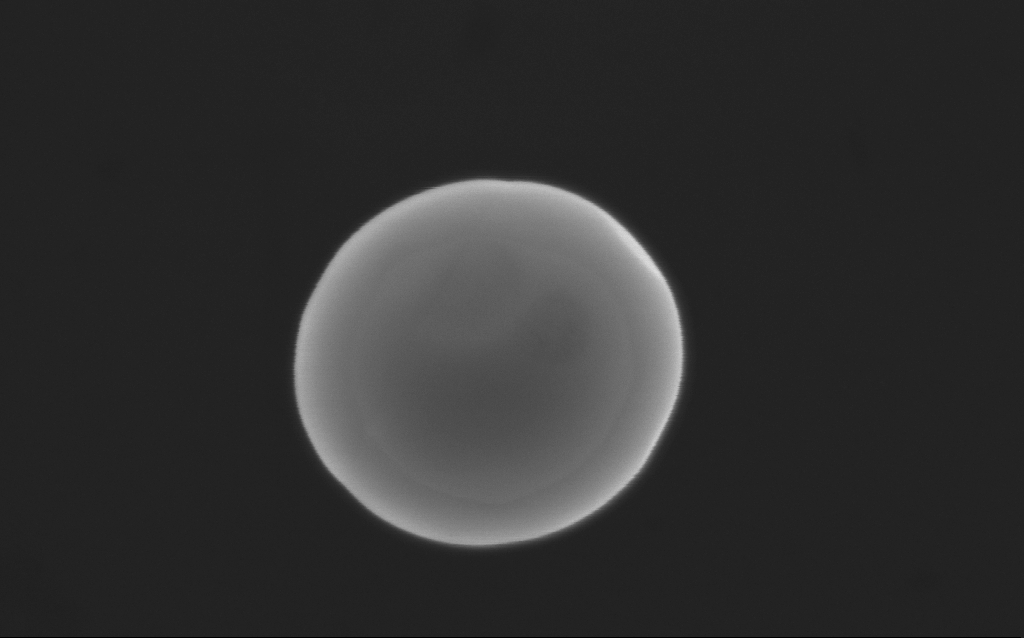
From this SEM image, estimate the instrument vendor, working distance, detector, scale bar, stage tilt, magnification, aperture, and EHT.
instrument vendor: Zeiss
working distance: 4 mm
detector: InLens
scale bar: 200 nm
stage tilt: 0°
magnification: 179.34 K X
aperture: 30 µm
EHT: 10 kV